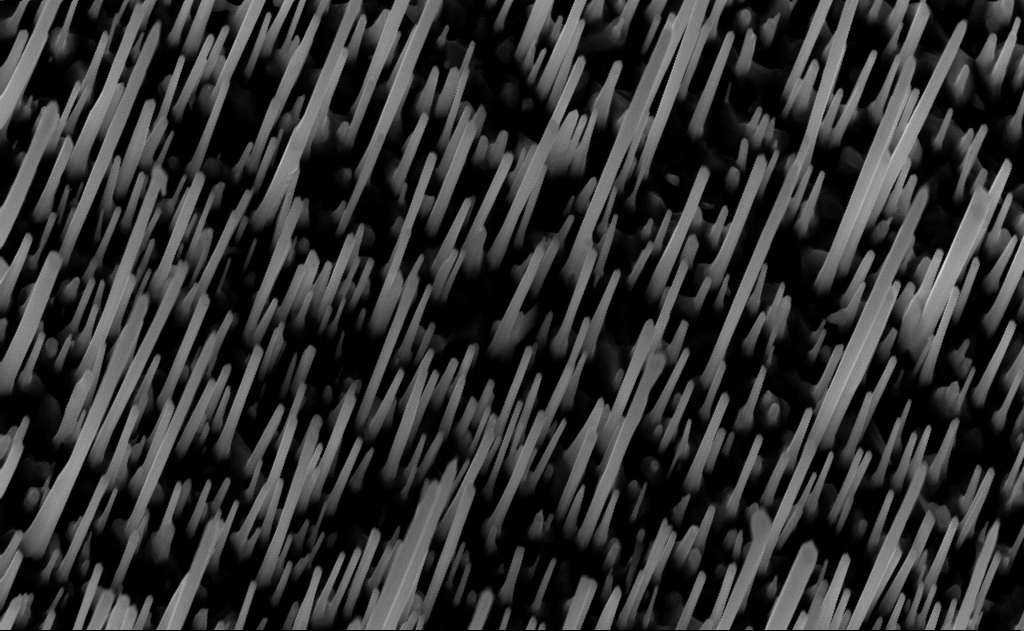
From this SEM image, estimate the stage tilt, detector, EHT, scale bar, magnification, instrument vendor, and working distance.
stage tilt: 0°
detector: InLens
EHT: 10 kV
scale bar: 1000 nm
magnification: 40 K X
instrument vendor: Zeiss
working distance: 7 mm